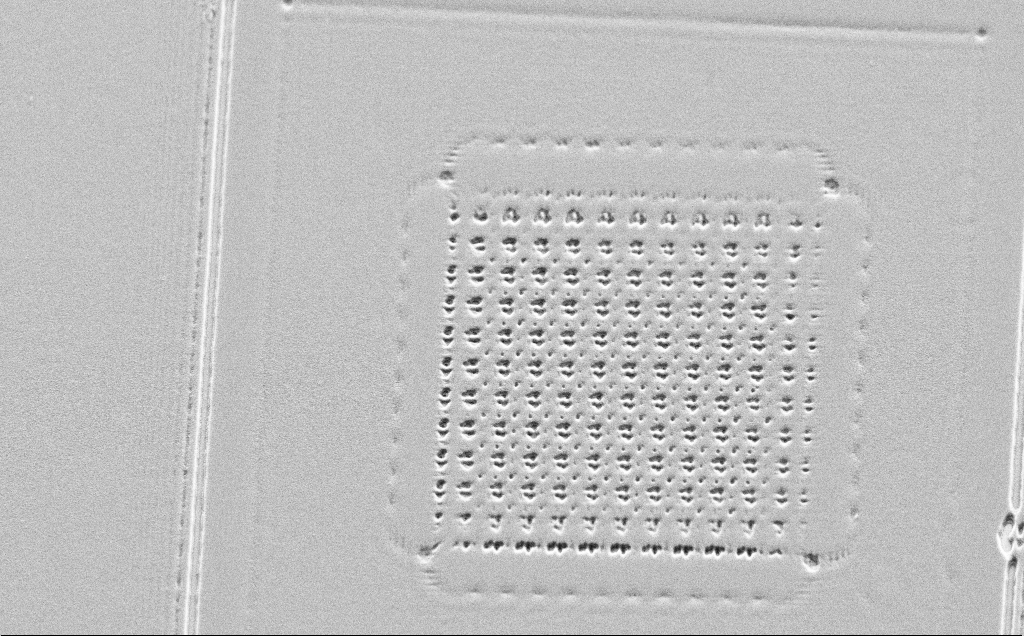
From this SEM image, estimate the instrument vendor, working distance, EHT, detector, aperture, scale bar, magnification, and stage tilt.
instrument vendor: Zeiss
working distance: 10 mm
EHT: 5 kV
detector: SE2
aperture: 30 µm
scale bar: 20000 nm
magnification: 2.89 K X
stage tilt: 45°